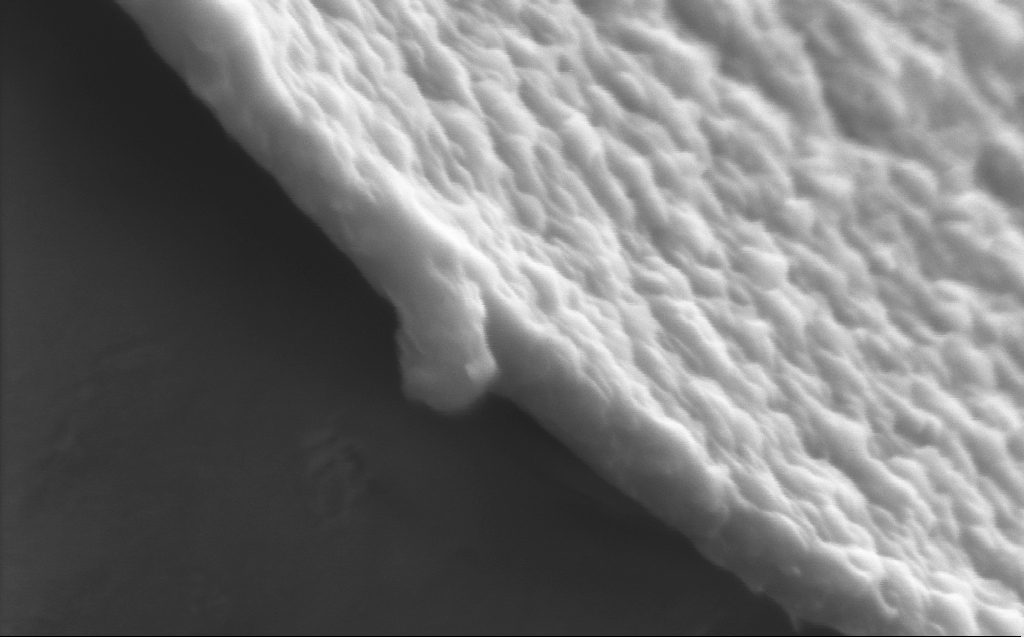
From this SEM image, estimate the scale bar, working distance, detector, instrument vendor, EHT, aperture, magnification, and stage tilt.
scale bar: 200 nm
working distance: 4 mm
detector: InLens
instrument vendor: Zeiss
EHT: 5 kV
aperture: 30 µm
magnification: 270.8 K X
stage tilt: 45°